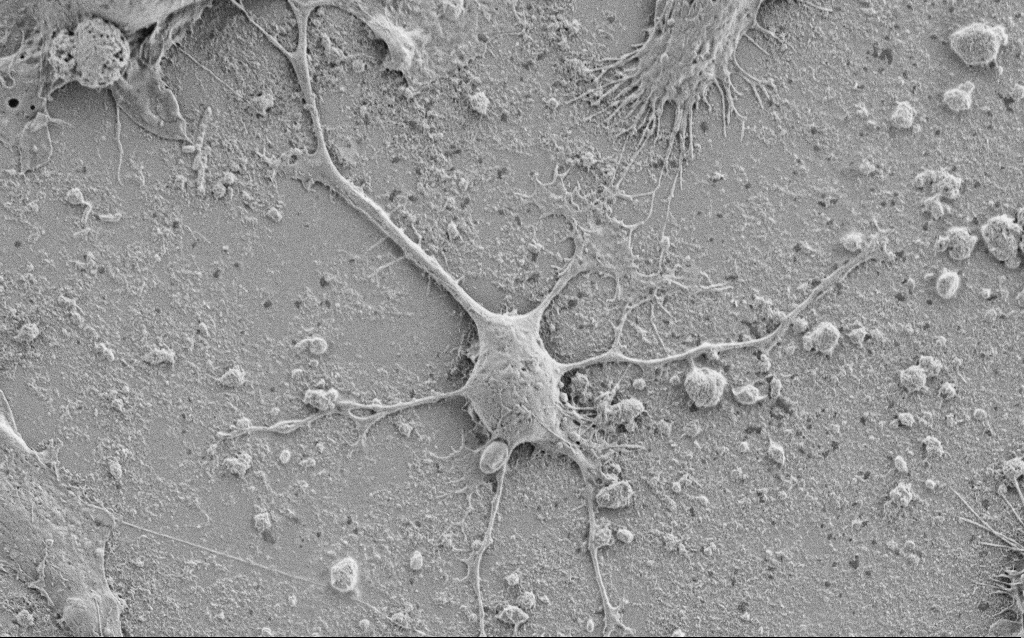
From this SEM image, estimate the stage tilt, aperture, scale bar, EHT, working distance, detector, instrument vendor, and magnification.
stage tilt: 0°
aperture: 30 µm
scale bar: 10000 nm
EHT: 2 kV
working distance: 6.9 mm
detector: SE2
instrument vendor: Zeiss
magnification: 3.5 K X